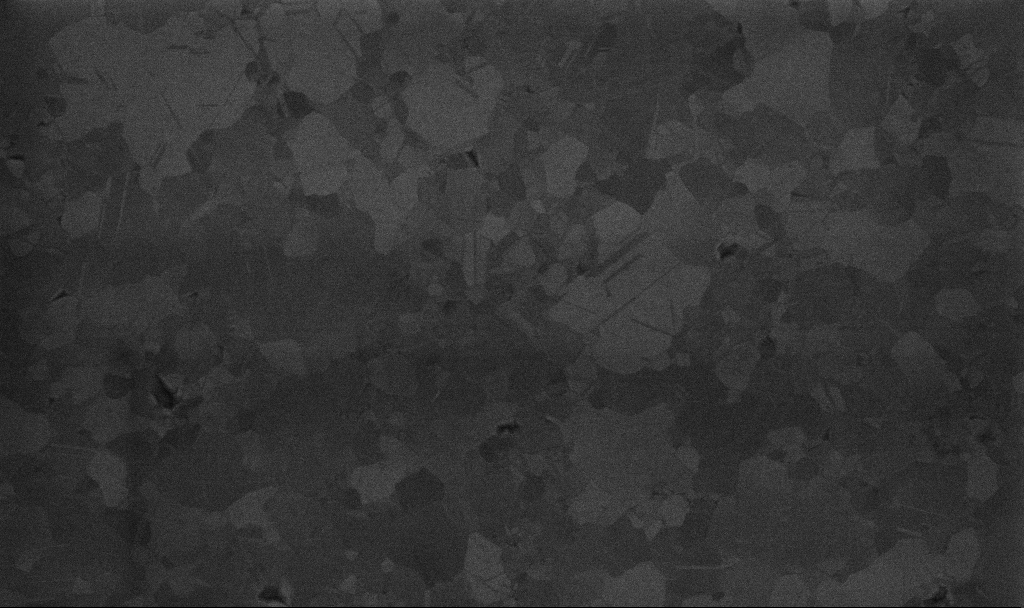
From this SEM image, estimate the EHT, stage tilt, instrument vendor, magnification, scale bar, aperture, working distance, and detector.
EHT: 10 kV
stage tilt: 0°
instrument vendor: Zeiss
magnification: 100 K X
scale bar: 200 nm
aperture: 30 µm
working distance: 3.4 mm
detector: InLens